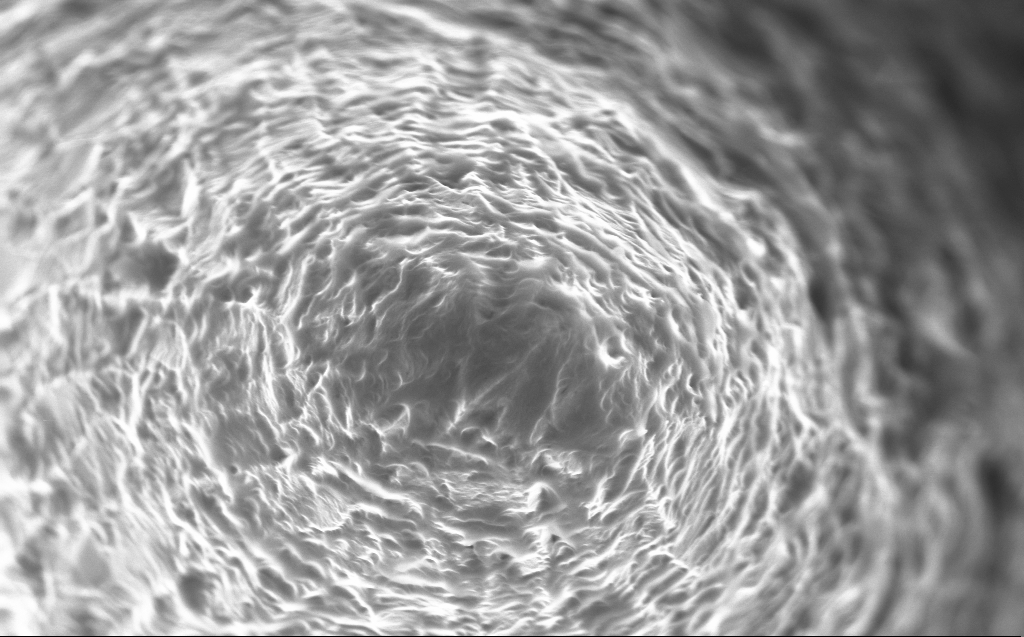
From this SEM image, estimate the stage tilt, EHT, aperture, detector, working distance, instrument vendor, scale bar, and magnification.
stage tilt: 0°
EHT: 10 kV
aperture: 30 µm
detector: InLens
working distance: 4 mm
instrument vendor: Zeiss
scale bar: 1000 nm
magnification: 57.58 K X